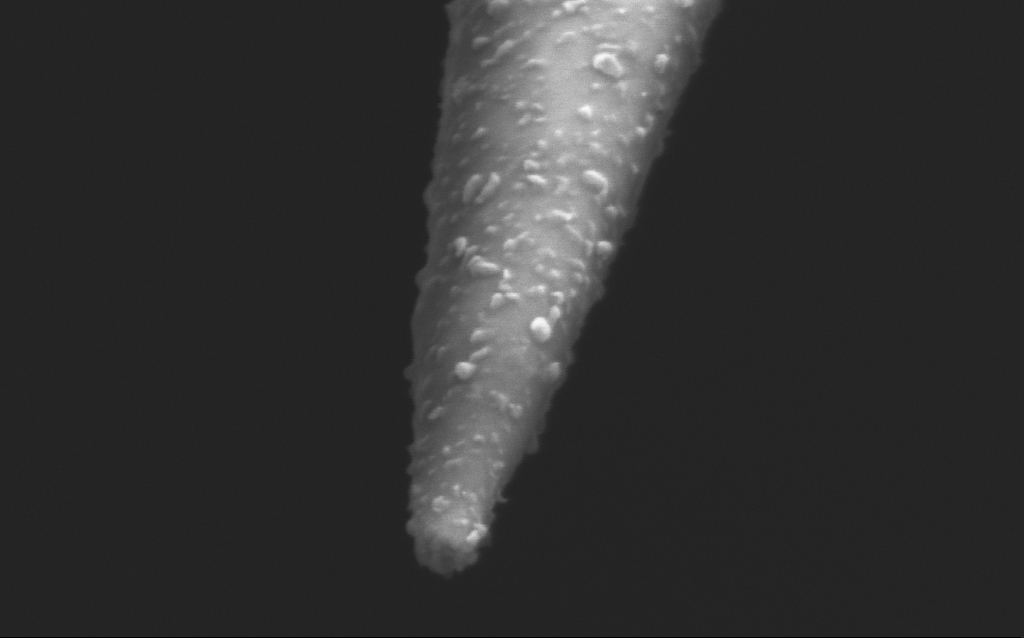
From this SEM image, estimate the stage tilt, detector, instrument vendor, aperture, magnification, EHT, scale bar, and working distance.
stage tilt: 45°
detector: InLens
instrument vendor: Zeiss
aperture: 30 µm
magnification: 250 K X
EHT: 2.5 kV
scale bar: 200 nm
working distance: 5 mm